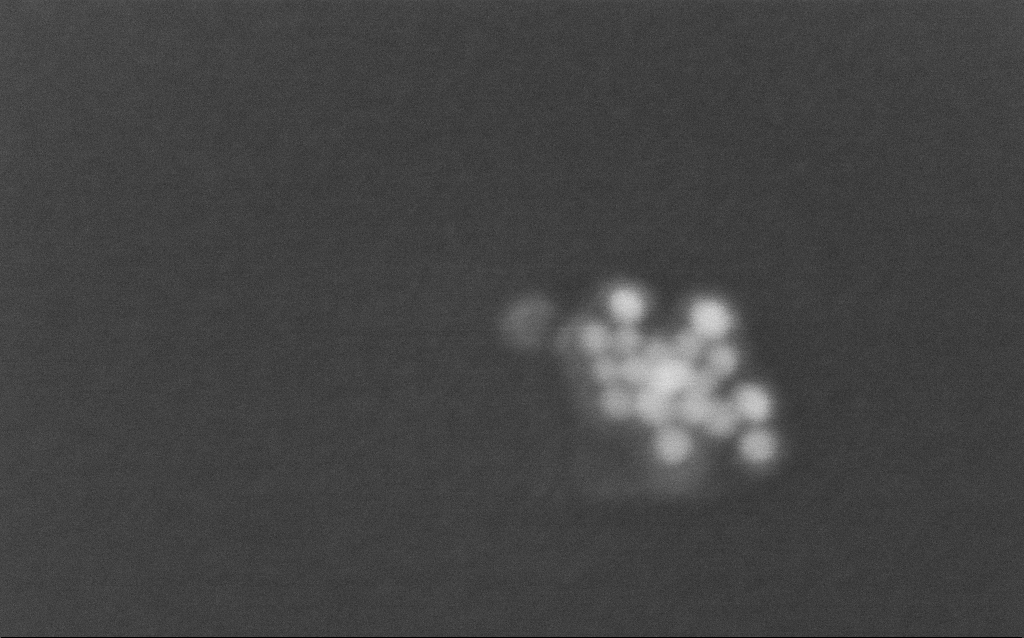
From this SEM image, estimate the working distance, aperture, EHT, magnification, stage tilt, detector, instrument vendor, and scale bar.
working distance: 2.9 mm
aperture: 30 µm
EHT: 4 kV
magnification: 909.53 K X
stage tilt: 0°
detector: InLens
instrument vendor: Zeiss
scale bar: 20 nm